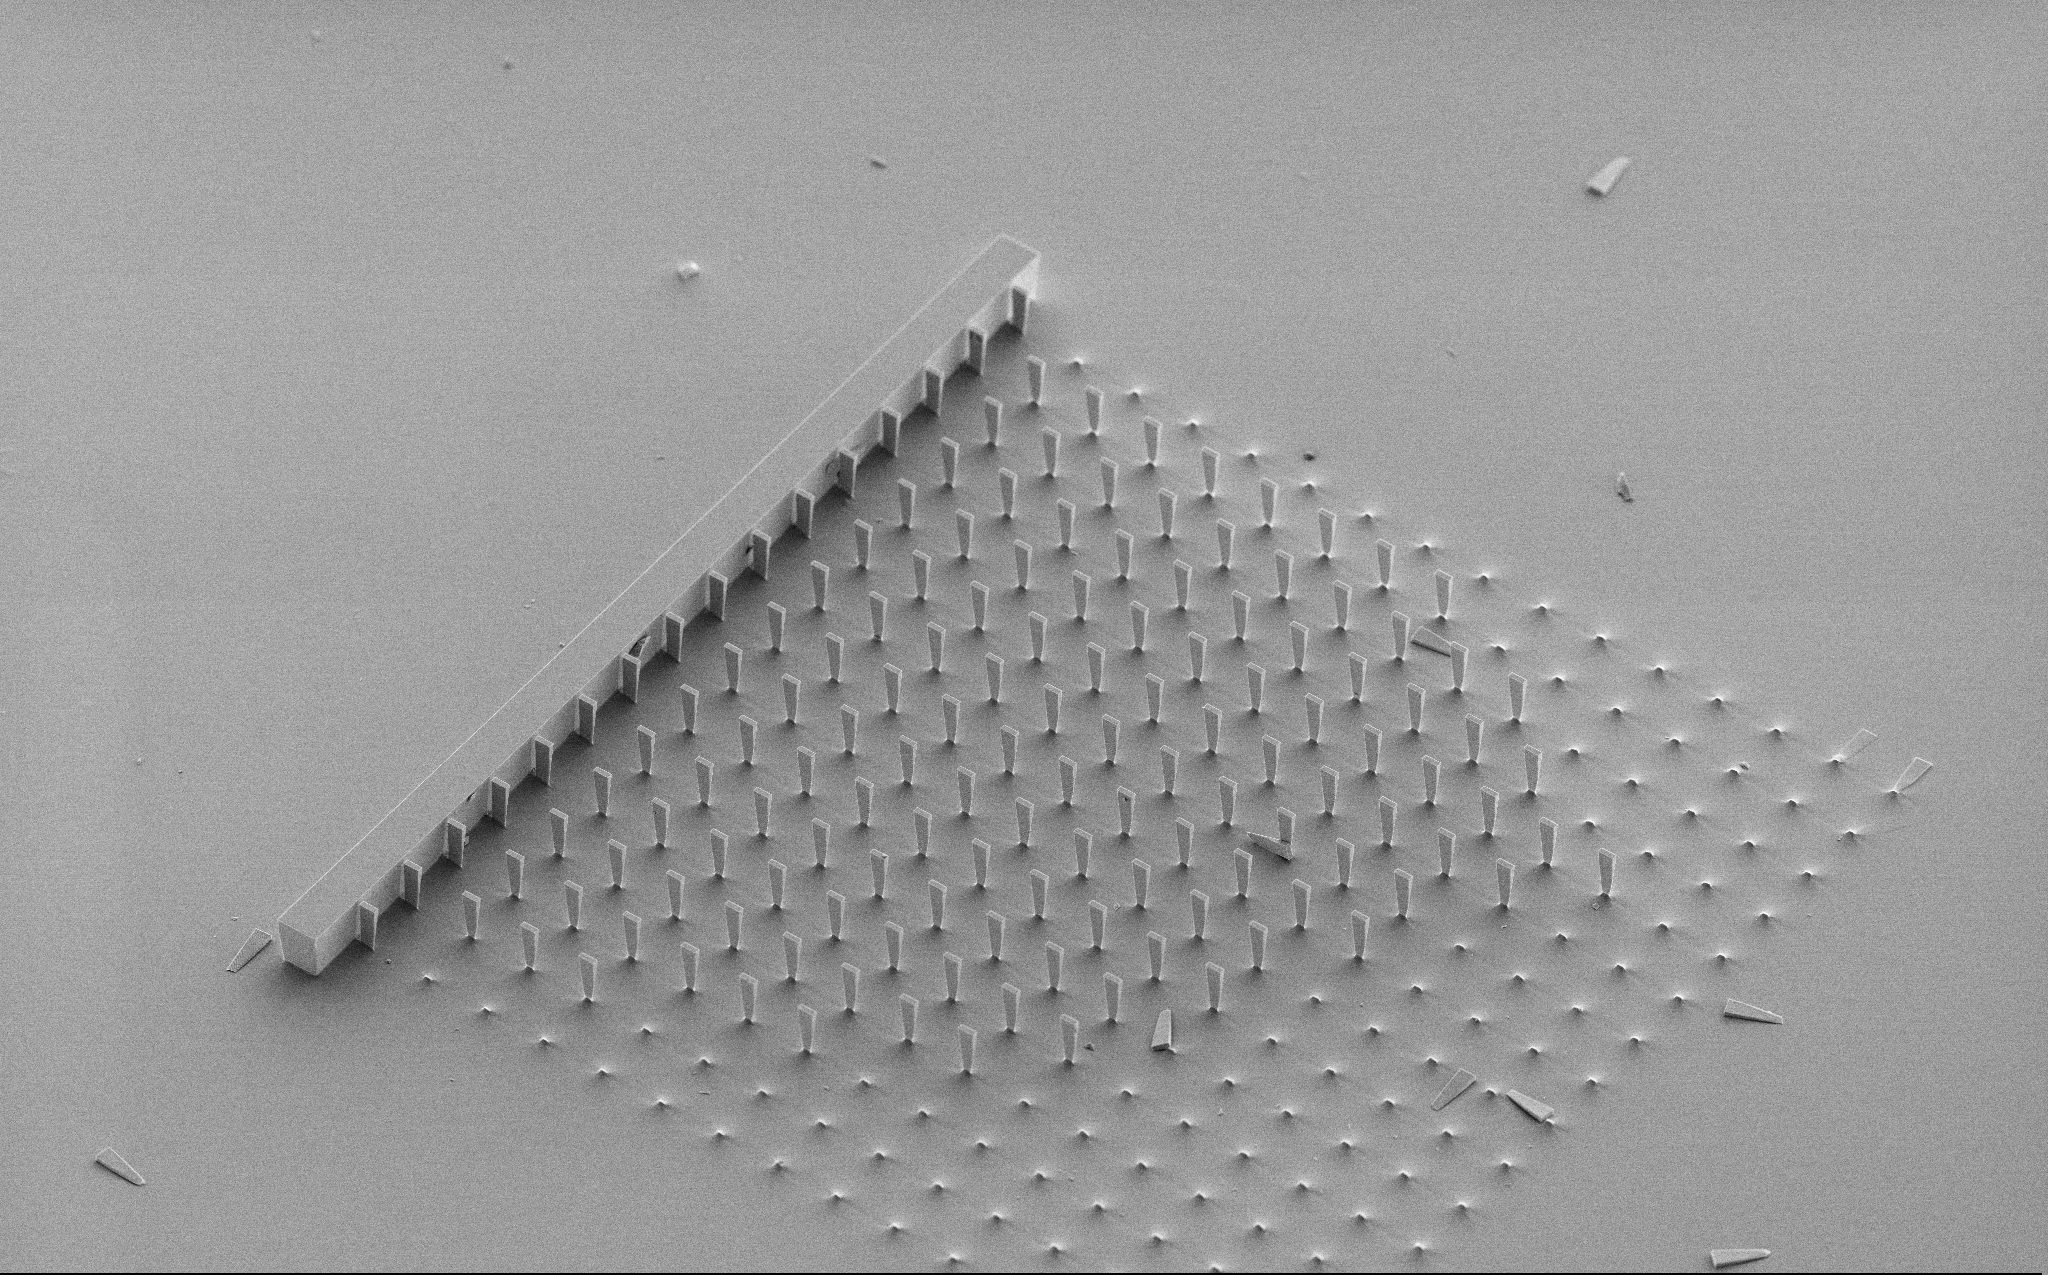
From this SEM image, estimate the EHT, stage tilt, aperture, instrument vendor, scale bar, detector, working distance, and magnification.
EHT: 5 kV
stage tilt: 45°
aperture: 30 µm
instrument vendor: Zeiss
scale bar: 100000 nm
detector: SE2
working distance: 10 mm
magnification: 0.447 K X